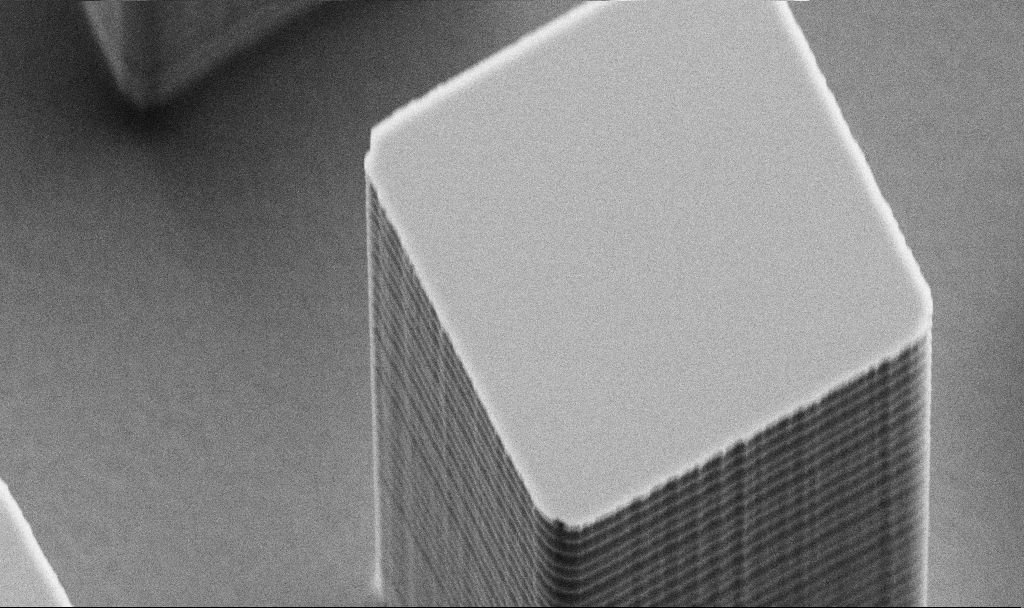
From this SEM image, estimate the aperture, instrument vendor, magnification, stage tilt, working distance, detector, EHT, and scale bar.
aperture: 30 µm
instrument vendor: Zeiss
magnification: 16.57 K X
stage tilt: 45°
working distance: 6.3 mm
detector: SE2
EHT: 5 kV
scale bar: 2000 nm